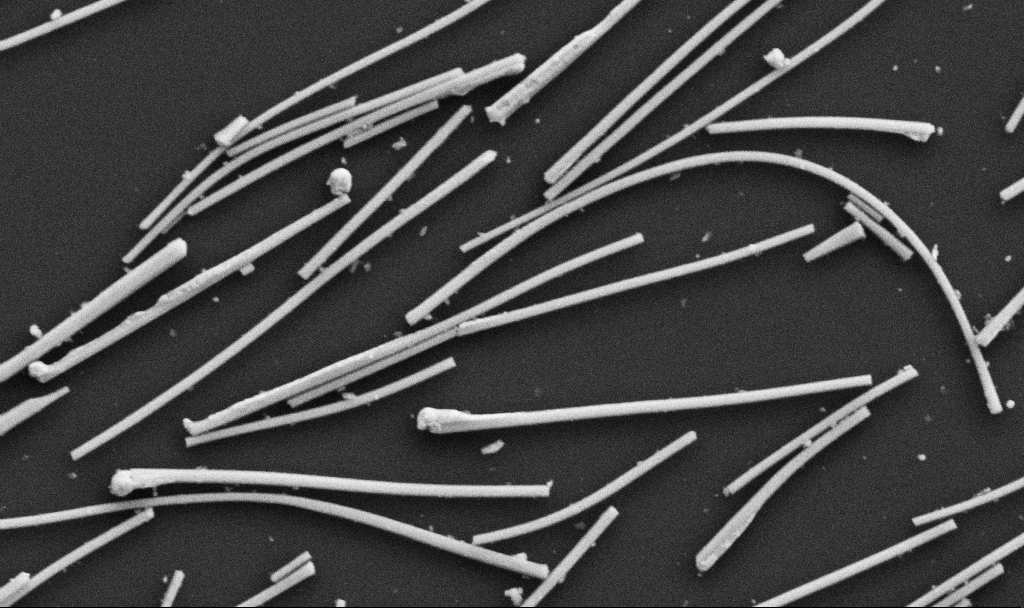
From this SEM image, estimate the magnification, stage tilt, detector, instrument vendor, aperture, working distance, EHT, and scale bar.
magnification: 37.92 K X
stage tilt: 0°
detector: SE2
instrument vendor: Zeiss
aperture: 30 µm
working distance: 10.7 mm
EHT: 5 kV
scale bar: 1000 nm